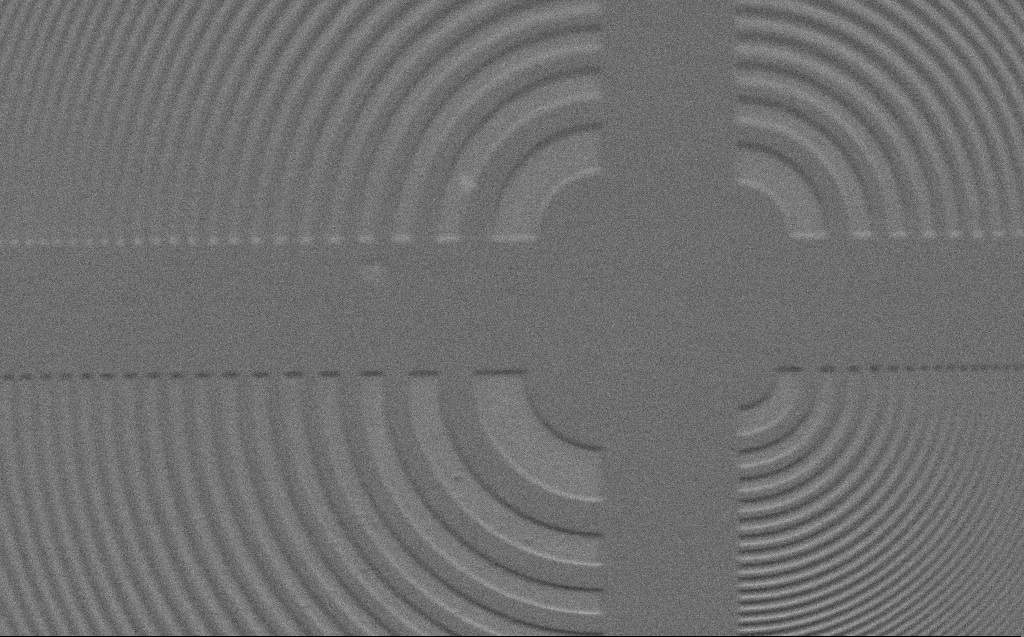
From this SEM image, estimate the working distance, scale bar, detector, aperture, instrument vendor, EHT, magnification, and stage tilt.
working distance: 4 mm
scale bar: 10000 nm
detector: SE2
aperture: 30 µm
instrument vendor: Zeiss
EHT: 2 kV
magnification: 5 K X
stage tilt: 45°